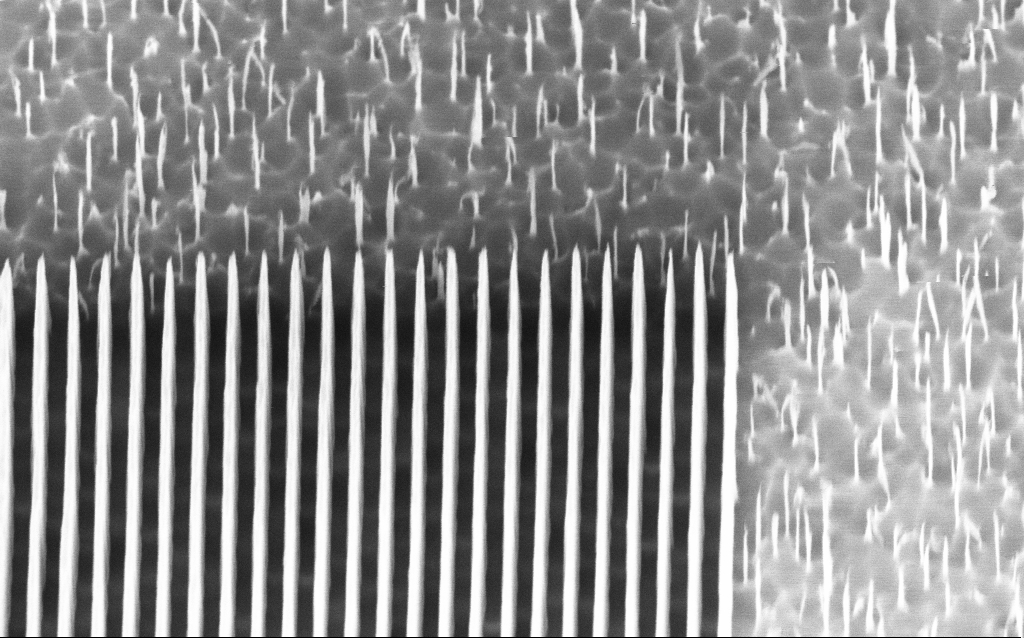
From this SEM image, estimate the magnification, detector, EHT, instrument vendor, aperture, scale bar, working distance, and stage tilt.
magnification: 56.63 K X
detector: InLens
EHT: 3 kV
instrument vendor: Zeiss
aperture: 30 µm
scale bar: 1000 nm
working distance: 5 mm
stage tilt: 45°